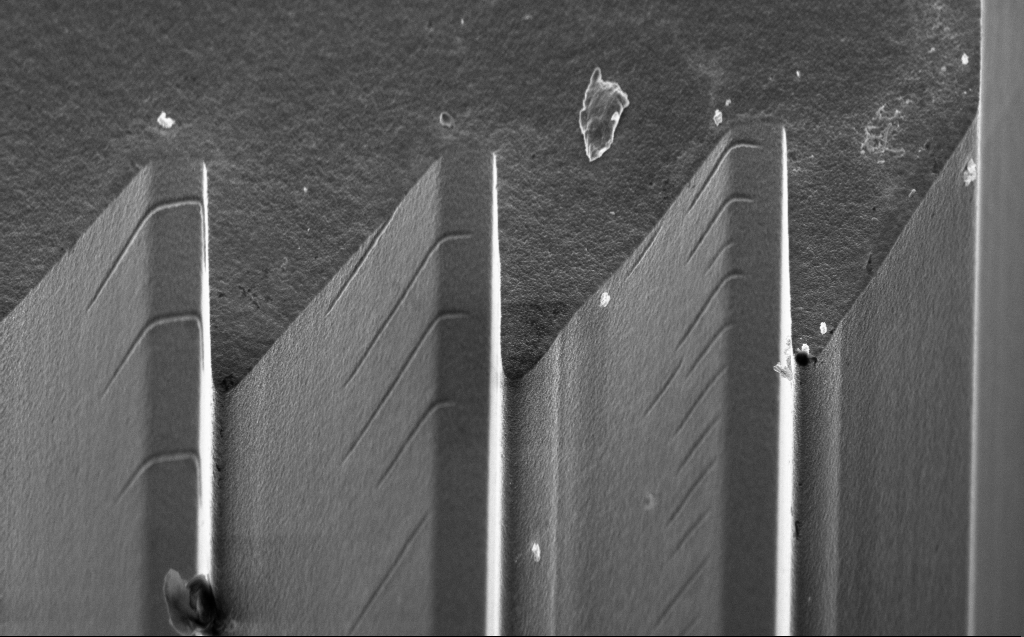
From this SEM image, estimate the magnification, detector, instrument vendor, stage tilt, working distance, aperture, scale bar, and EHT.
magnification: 9.44 K X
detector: InLens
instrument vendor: Zeiss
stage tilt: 45°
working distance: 5 mm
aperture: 30 µm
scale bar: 2000 nm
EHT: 5 kV